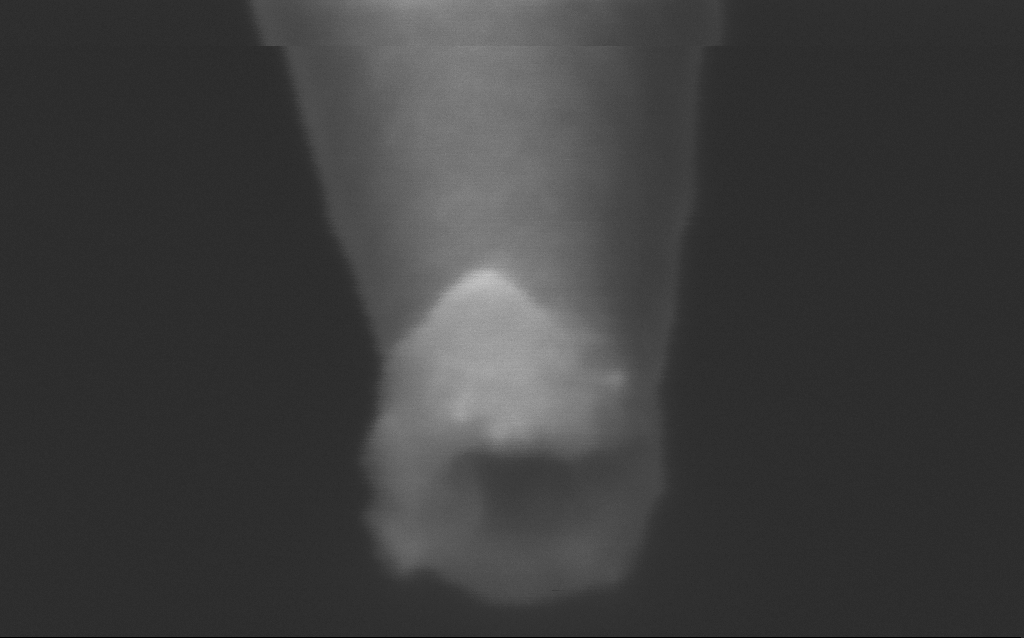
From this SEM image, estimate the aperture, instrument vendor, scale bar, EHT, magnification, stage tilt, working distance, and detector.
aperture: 30 µm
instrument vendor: Zeiss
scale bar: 20 nm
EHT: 2.5 kV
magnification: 1000 K X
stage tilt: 45°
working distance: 5 mm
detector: InLens